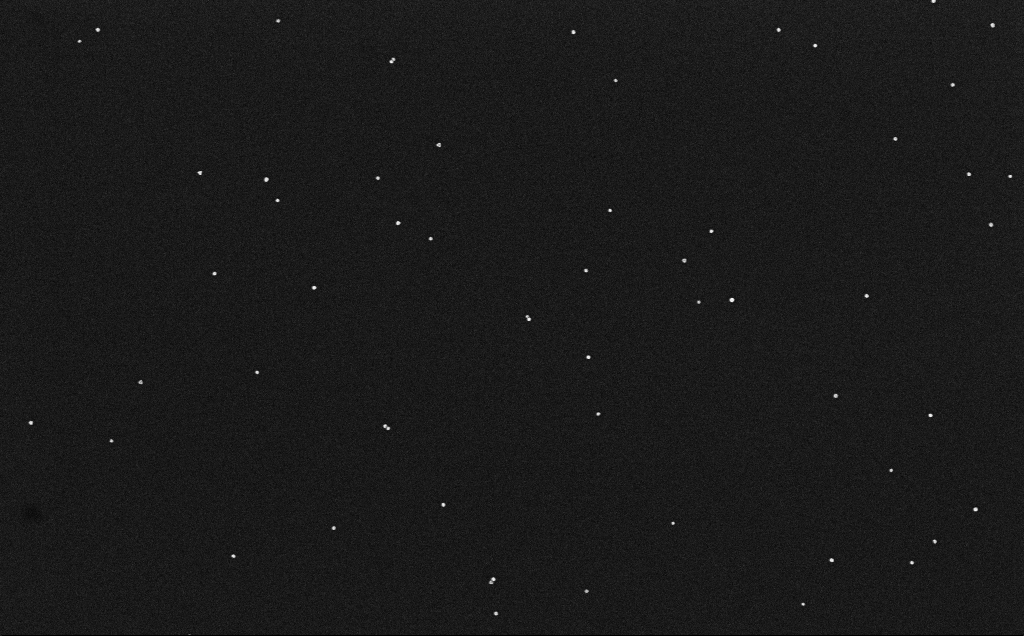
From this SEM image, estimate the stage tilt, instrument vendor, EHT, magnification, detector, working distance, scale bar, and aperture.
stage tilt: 0°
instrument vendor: Zeiss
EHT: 10 kV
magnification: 100 K X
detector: InLens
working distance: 3.2 mm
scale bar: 200 nm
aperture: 30 µm